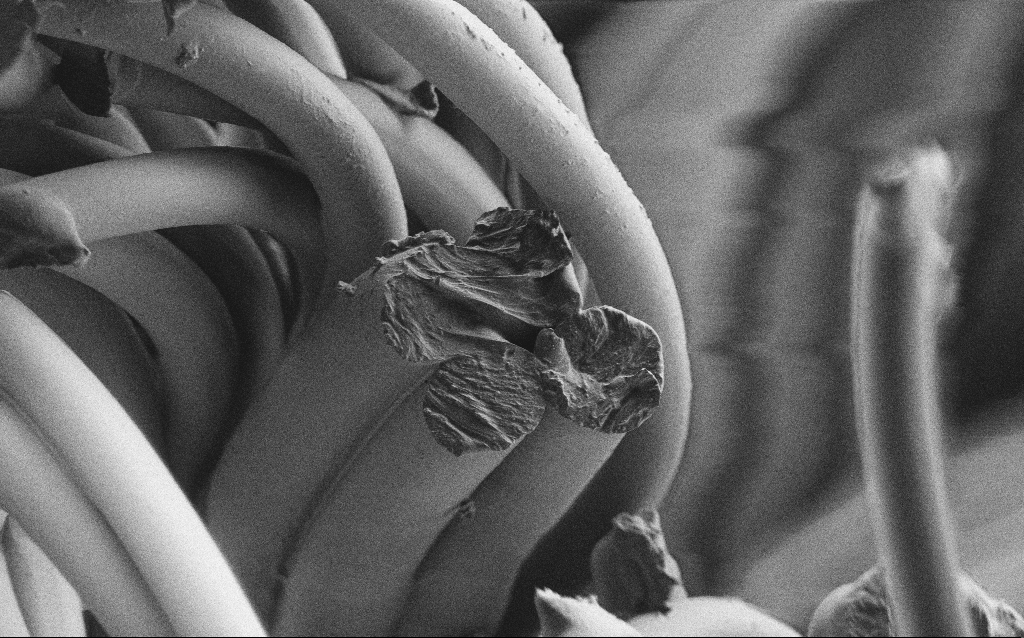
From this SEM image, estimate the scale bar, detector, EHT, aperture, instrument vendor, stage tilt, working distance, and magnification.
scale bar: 20000 nm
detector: SE2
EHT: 1 kV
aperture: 30 µm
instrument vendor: Zeiss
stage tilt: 0°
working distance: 3 mm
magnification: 1.23 K X